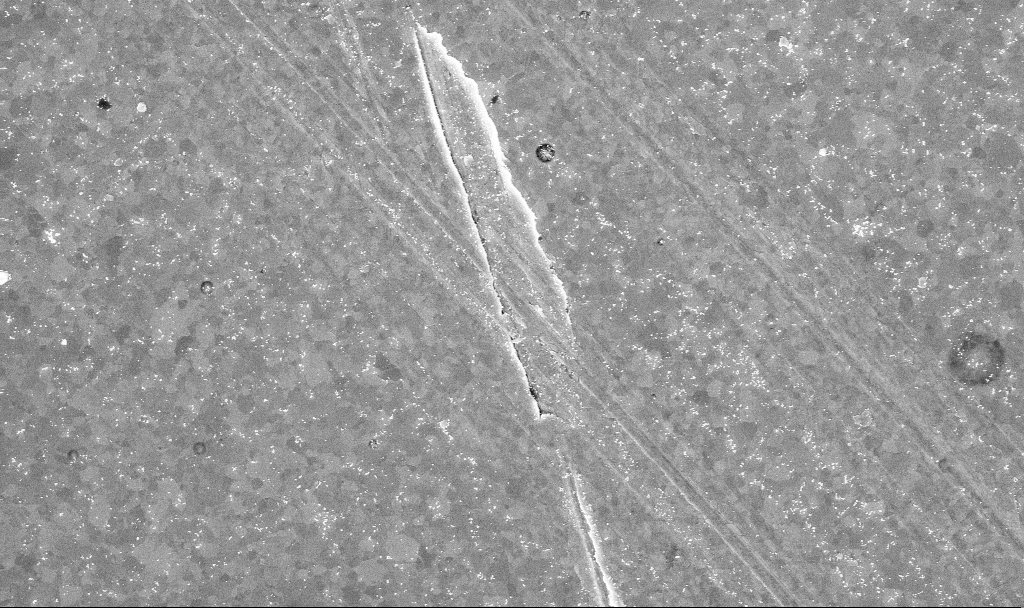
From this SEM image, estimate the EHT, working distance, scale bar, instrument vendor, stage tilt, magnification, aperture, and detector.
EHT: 10 kV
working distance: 3.4 mm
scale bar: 1000 nm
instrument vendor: Zeiss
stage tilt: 0°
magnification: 20.42 K X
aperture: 30 µm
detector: InLens